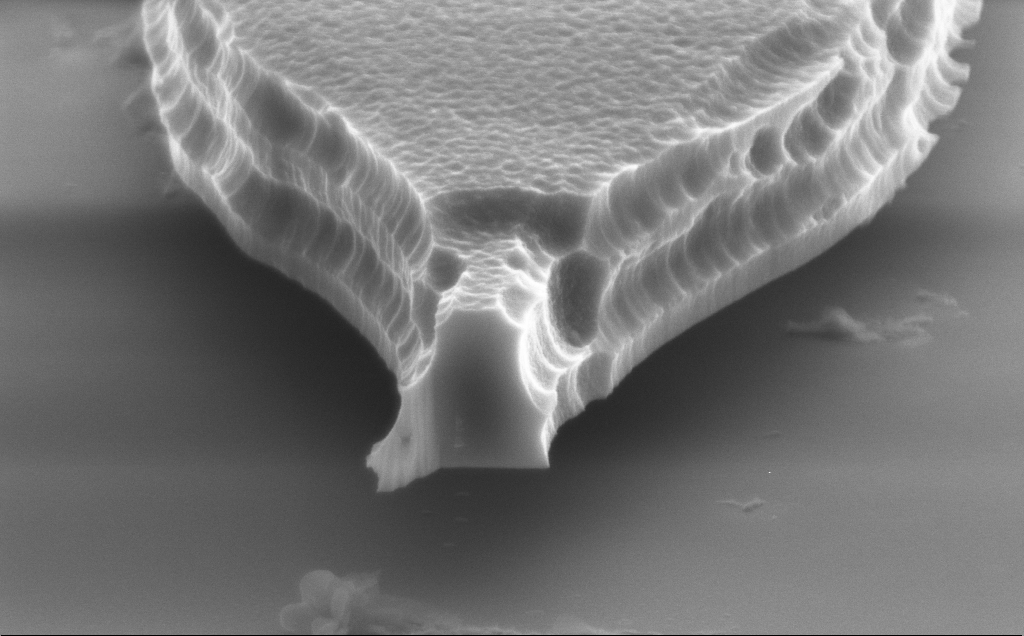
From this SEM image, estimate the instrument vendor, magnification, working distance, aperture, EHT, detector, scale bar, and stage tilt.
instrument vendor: Zeiss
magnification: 36.72 K X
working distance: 12 mm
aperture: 30 µm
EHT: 8 kV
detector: SE2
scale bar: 1000 nm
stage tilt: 70°